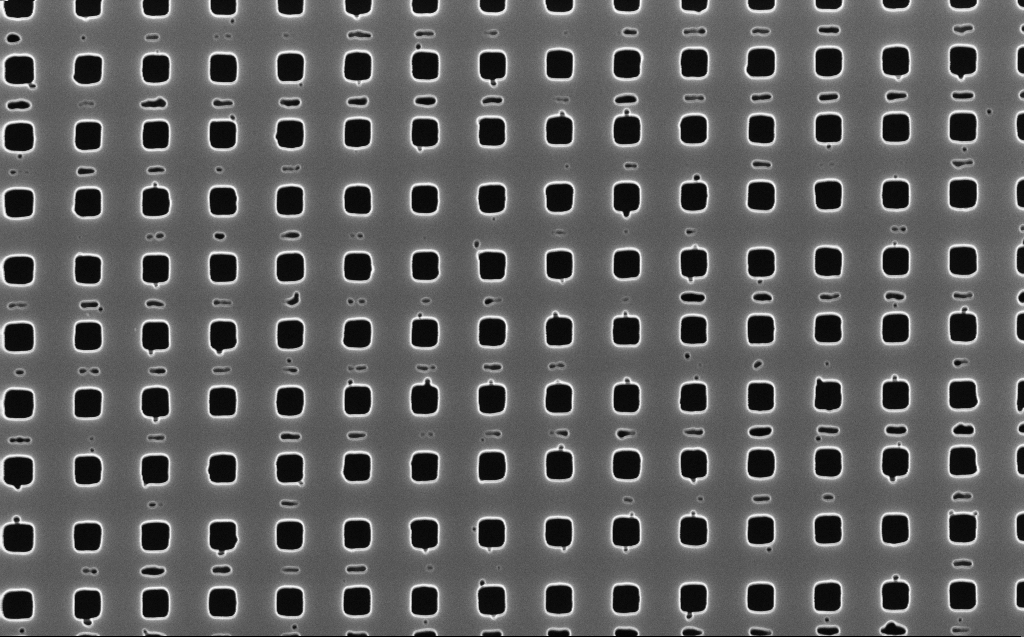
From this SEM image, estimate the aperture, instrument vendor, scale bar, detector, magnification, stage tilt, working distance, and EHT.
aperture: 30 µm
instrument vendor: Zeiss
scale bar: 1000 nm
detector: InLens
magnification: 50 K X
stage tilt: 0°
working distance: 5 mm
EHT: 10 kV